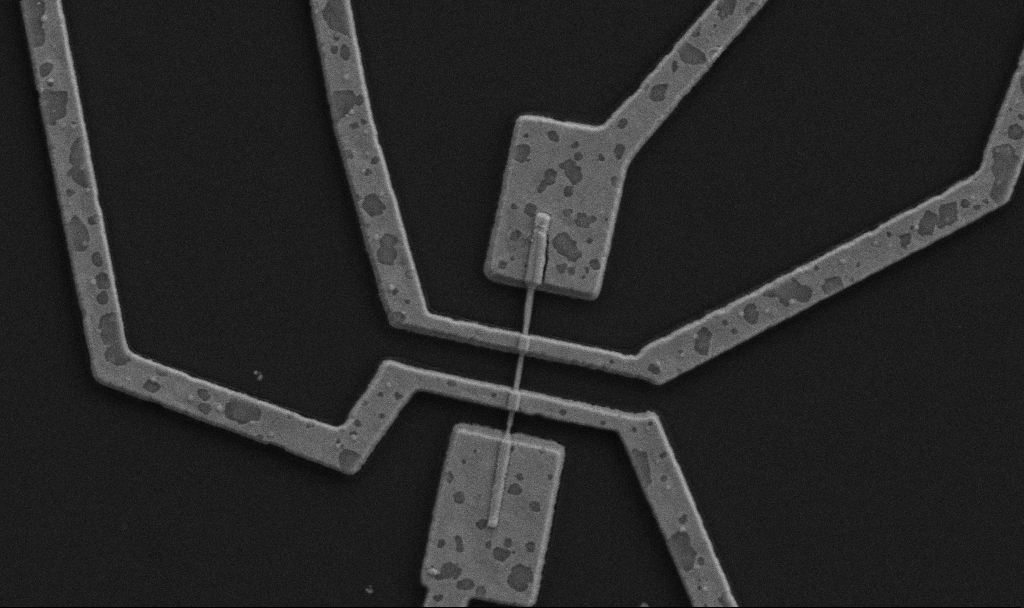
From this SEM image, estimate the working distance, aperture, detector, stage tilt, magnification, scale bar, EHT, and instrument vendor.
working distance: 10.7 mm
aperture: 30 µm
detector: SE2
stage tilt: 0°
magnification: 20 K X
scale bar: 1000 nm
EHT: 5 kV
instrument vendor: Zeiss